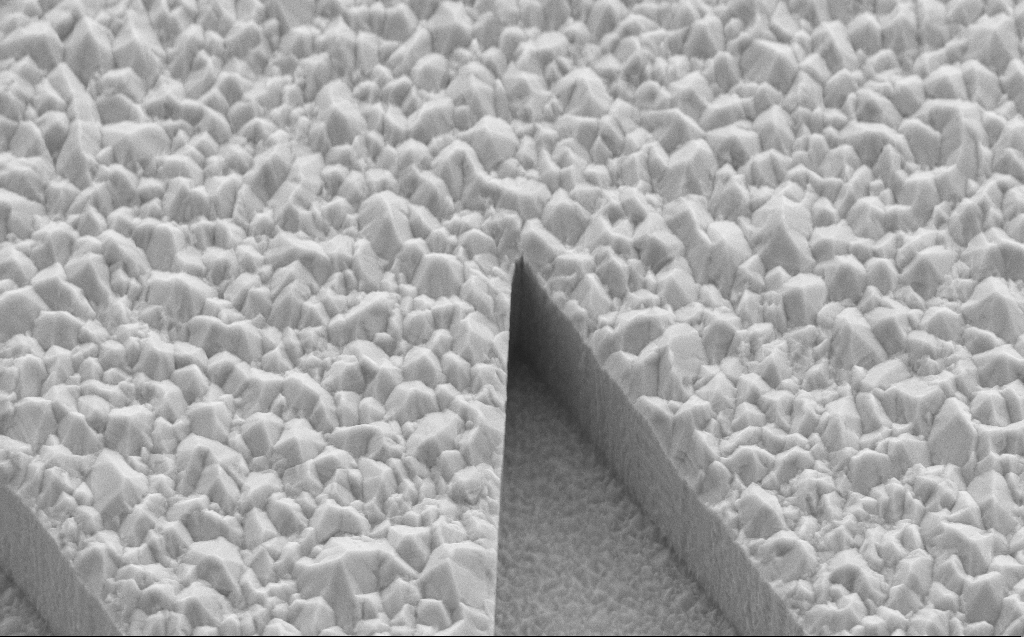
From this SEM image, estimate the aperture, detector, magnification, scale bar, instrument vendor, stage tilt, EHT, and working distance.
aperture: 30 µm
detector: SE2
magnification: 22.63 K X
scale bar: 1000 nm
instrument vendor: Zeiss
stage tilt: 45°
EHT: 10 kV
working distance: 5 mm